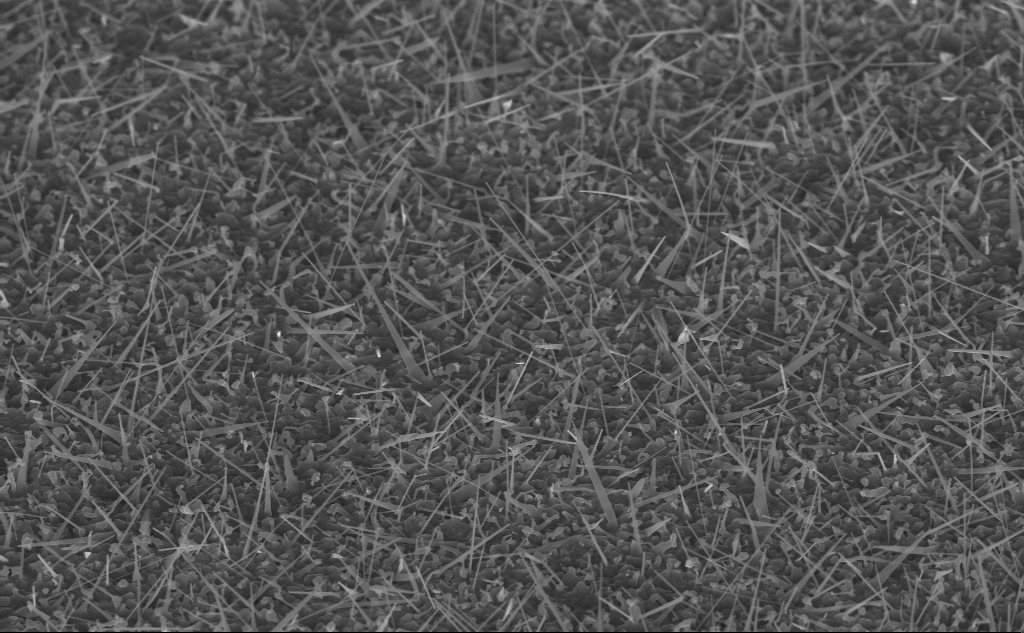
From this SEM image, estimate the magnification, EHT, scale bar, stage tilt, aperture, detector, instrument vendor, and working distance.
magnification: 20 K X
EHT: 10 kV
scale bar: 2000 nm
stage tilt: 45°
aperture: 30 µm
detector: InLens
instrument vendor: Zeiss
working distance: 8 mm